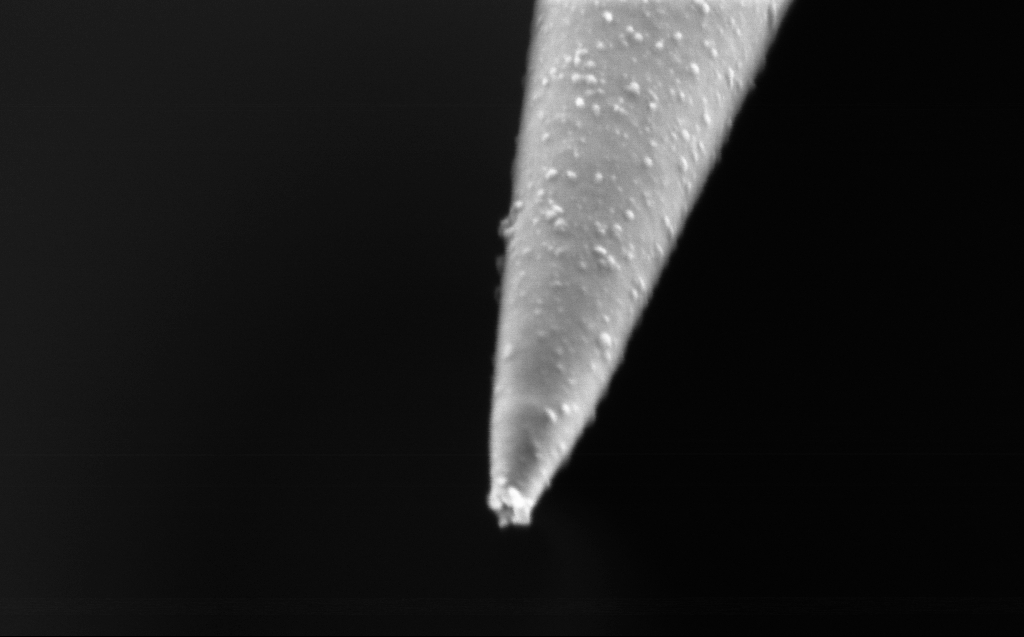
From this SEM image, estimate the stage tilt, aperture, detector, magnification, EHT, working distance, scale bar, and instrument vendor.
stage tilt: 45°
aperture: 30 µm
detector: InLens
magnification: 137.05 K X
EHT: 1 kV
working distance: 3 mm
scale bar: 200 nm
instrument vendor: Zeiss